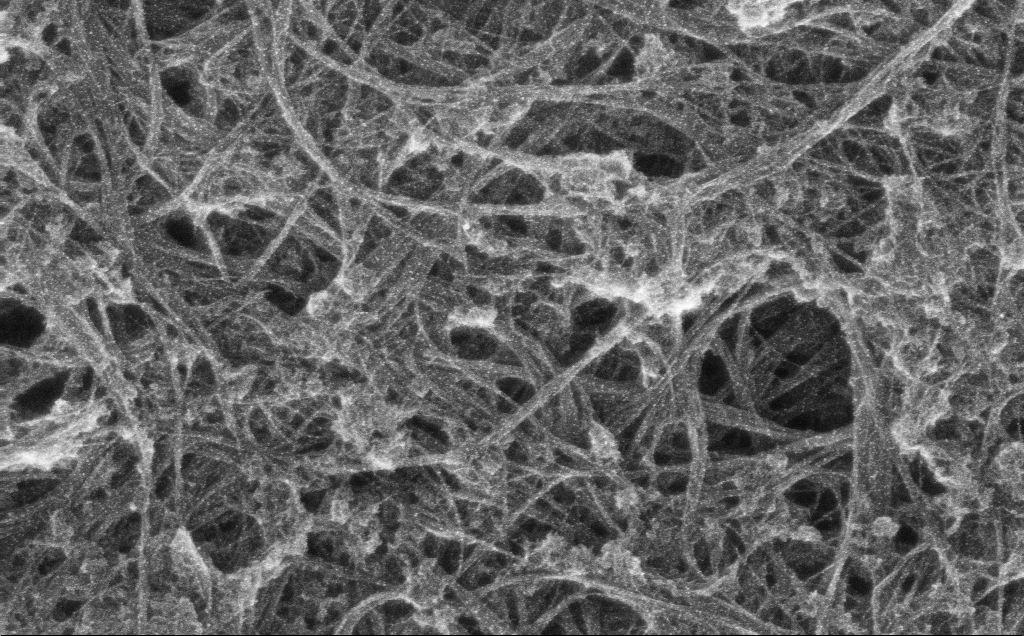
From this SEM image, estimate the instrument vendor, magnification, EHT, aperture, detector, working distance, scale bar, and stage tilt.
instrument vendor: Zeiss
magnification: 109.89 K X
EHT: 10 kV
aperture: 30 µm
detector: InLens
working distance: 3 mm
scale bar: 200 nm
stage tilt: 0°